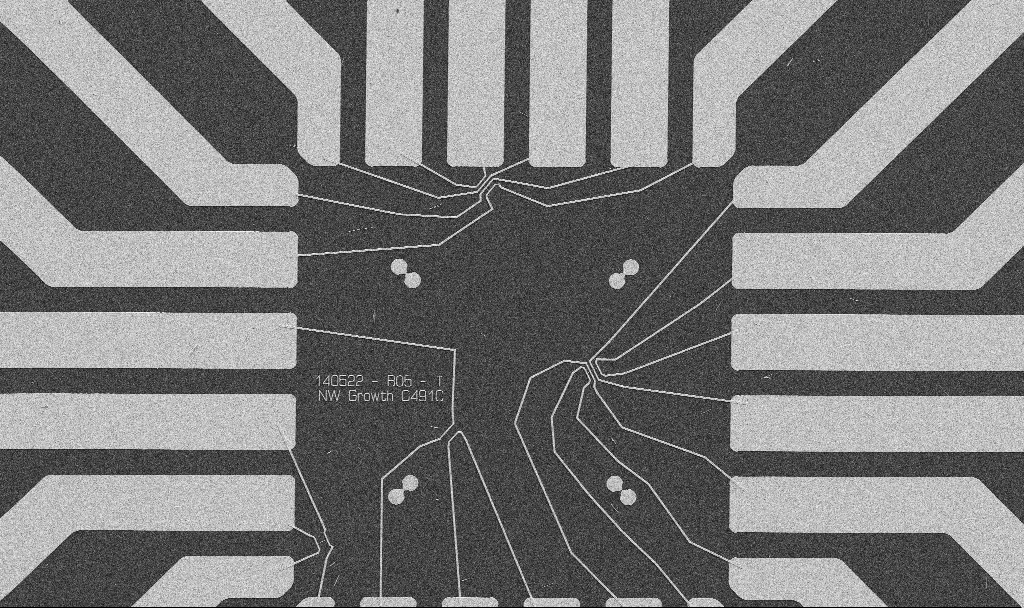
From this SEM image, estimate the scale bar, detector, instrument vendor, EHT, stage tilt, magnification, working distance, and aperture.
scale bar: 20000 nm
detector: SE2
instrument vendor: Zeiss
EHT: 5 kV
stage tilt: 0°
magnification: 1 K X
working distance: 10.7 mm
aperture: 30 µm